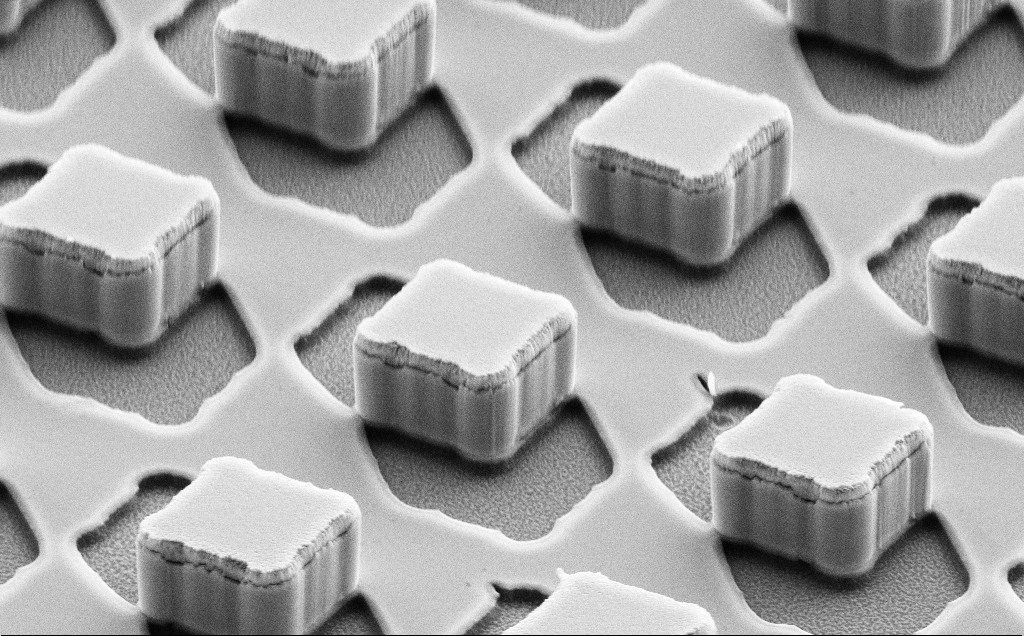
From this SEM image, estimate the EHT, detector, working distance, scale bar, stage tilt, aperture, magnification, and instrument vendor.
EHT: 10 kV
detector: SE2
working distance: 10 mm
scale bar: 2000 nm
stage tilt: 55.6°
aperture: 30 µm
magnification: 7.67 K X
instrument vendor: Zeiss